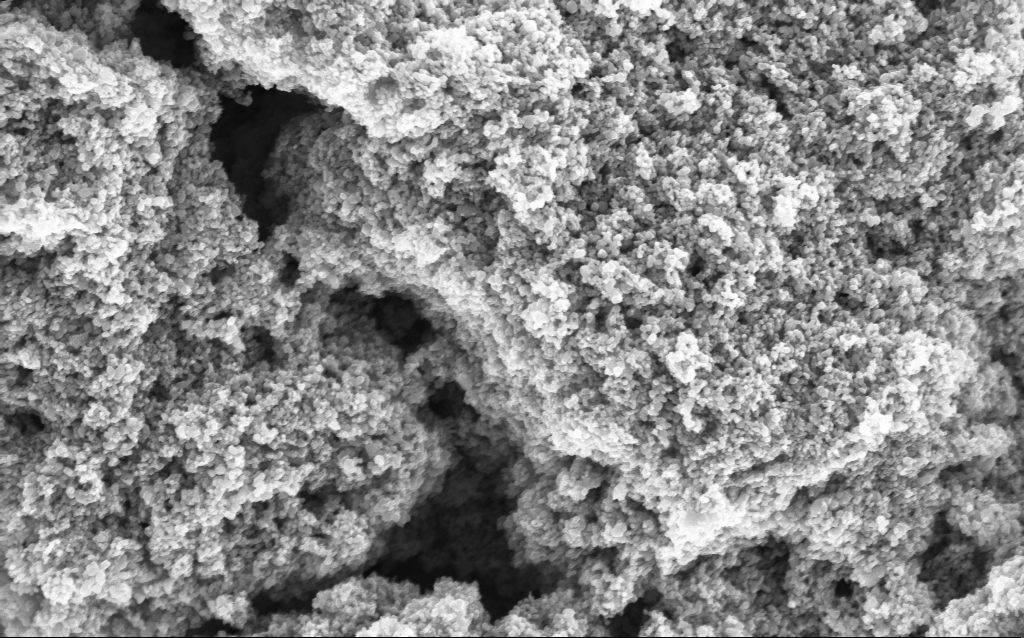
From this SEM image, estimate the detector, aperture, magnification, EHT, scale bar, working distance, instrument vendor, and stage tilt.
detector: InLens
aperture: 30 µm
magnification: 68.65 K X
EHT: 5 kV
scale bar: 1000 nm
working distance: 4.7 mm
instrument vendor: Zeiss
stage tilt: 0°